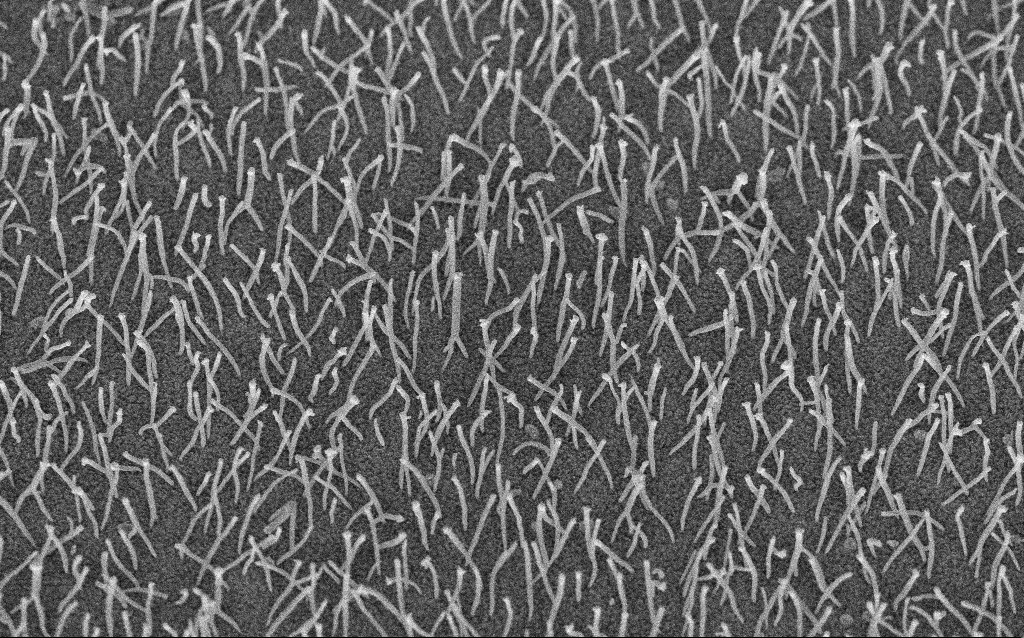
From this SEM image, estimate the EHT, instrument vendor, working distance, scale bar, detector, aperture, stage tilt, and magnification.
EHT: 5 kV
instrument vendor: Zeiss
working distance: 8.3 mm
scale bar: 1000 nm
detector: InLens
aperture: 30 µm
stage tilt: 45°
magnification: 20 K X